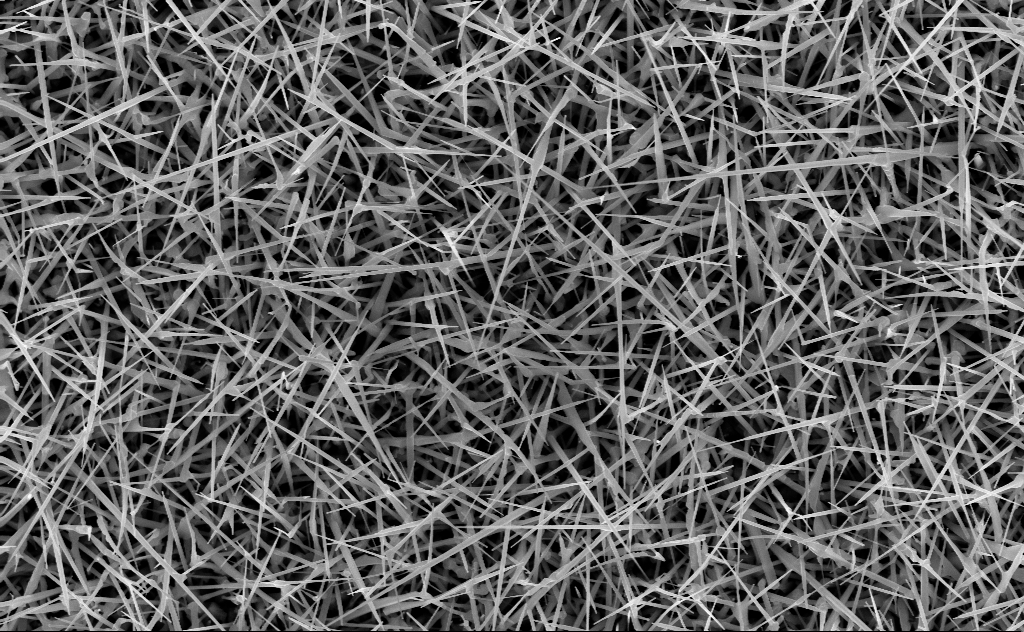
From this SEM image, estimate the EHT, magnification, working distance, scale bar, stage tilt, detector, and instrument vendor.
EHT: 10 kV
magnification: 20 K X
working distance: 10 mm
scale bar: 1000 nm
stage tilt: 0°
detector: InLens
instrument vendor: Zeiss